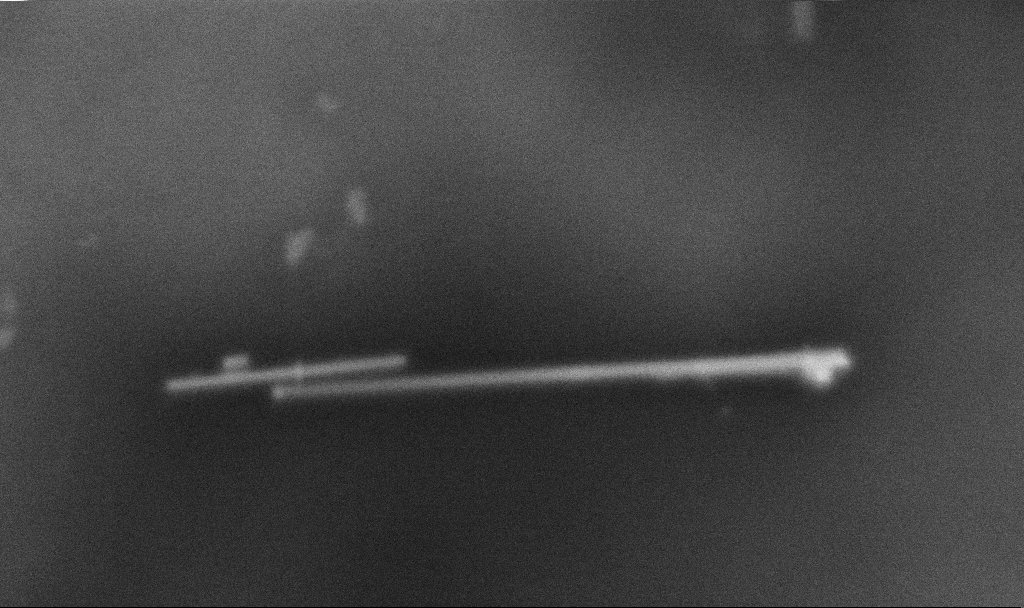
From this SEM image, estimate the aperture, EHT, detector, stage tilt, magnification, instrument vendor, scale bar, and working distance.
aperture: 30 µm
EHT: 3 kV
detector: InLens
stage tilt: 0°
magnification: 154.88 K X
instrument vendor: Zeiss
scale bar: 200 nm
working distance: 3.3 mm